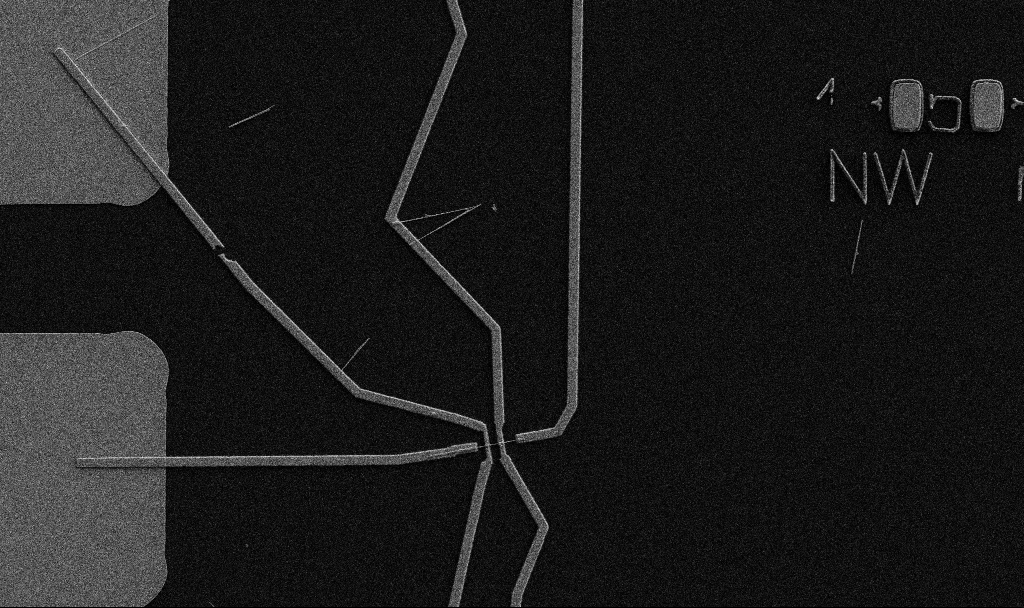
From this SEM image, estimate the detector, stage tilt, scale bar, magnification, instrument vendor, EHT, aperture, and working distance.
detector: SE2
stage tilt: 0°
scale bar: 10000 nm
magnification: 5 K X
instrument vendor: Zeiss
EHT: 5 kV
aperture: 30 µm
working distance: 10.7 mm